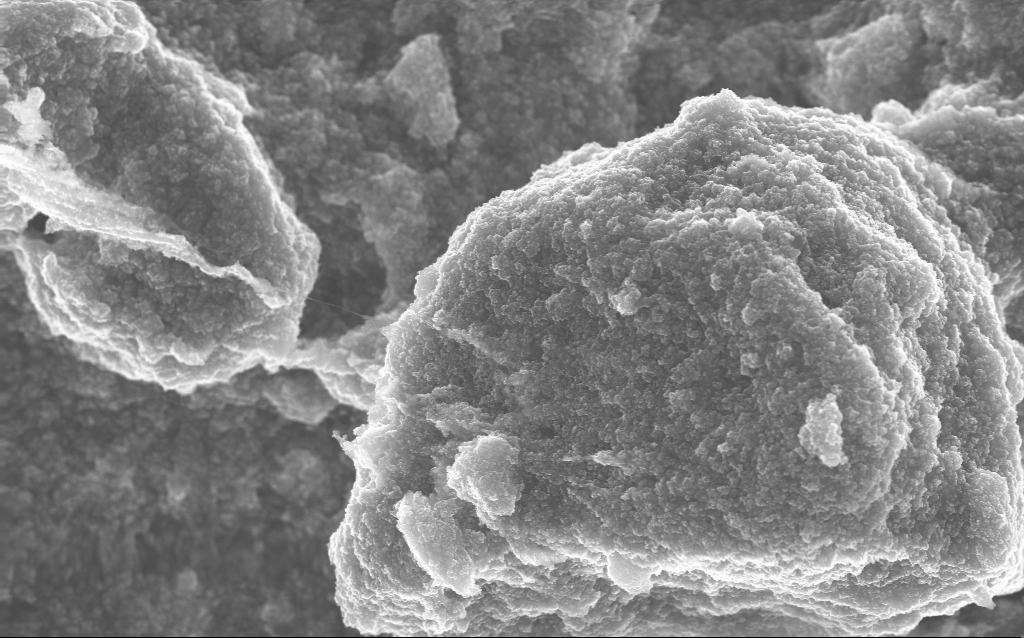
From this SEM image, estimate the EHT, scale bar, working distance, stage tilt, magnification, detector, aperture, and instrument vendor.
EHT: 10 kV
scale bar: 1000 nm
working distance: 2.7 mm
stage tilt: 0°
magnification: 20.87 K X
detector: InLens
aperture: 30 µm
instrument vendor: Zeiss